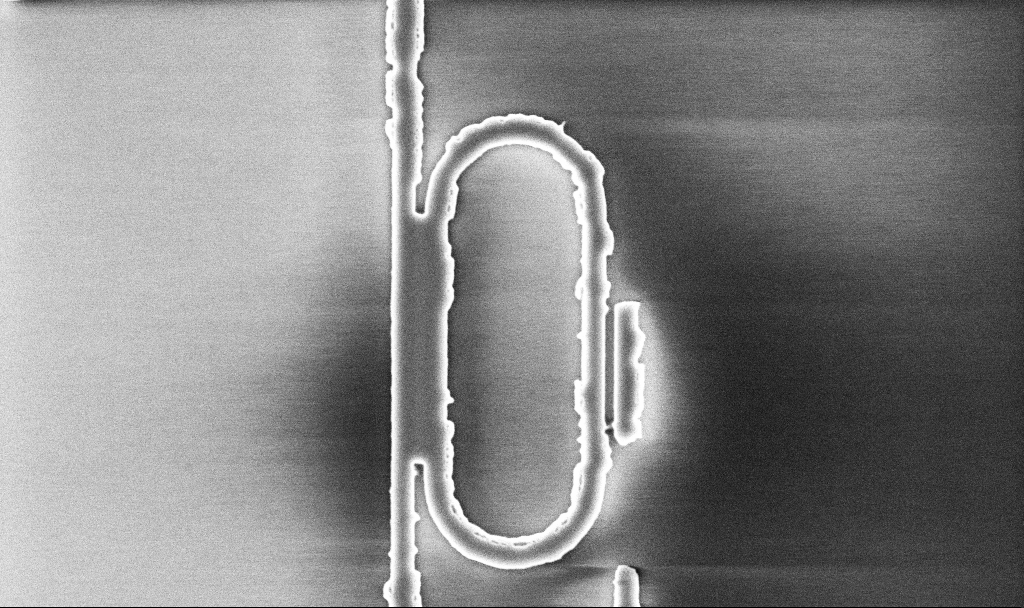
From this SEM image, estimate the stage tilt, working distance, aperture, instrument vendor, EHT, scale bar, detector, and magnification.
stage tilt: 0°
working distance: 10.1 mm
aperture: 30 µm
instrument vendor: Zeiss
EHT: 5 kV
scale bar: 2000 nm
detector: InLens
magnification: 16.67 K X